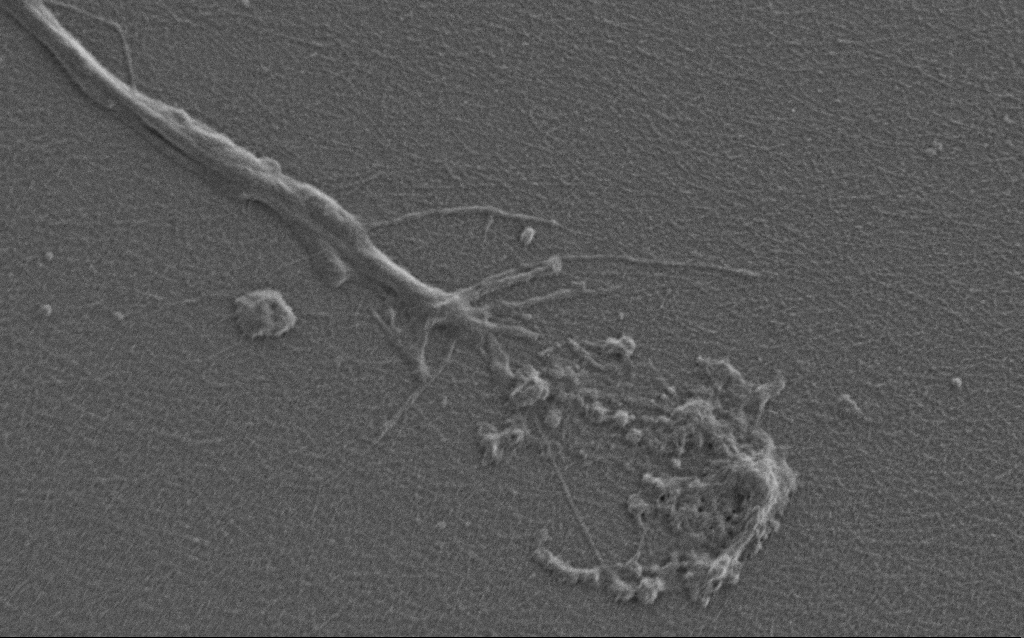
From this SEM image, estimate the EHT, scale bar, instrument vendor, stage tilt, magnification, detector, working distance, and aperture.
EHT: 0.9 kV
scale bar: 2000 nm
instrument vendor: Zeiss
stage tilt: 0°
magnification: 10 K X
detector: SE2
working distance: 7 mm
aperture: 30 µm